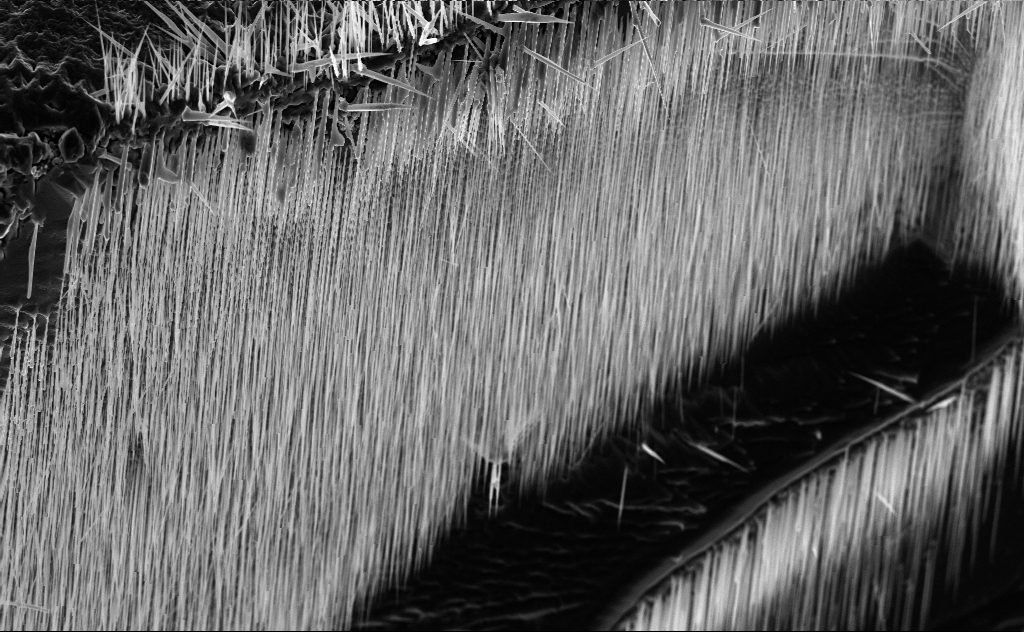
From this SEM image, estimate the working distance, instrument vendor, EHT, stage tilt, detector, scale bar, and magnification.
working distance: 6 mm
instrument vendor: Zeiss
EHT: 10 kV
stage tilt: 45°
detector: InLens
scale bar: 10000 nm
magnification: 4.38 K X